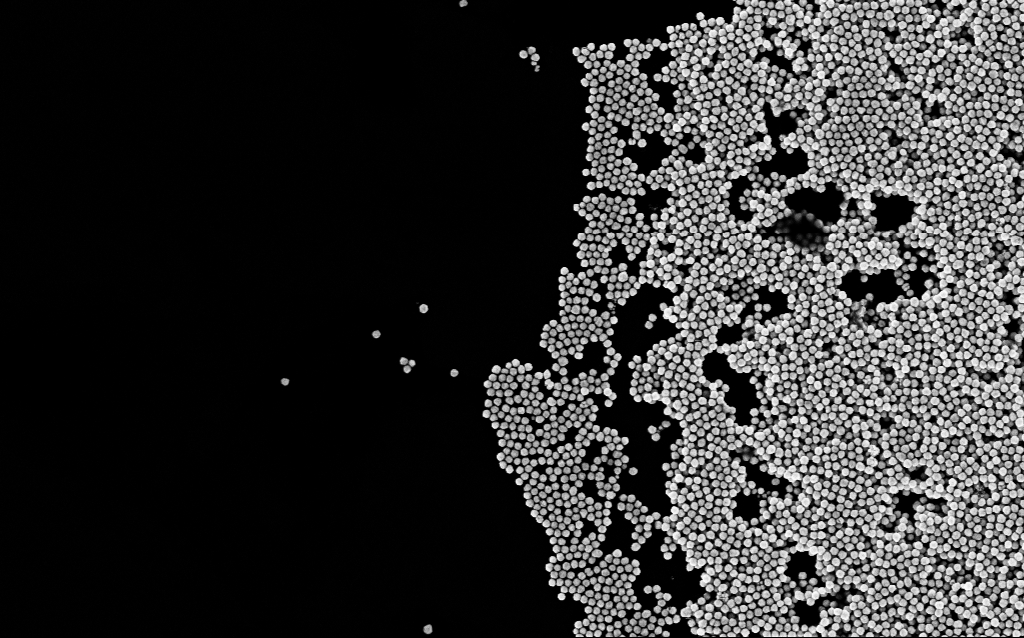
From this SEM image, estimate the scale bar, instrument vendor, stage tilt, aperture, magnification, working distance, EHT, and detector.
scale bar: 1000 nm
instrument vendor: Zeiss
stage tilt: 0°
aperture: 30 µm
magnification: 50 K X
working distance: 3.3 mm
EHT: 5 kV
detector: InLens